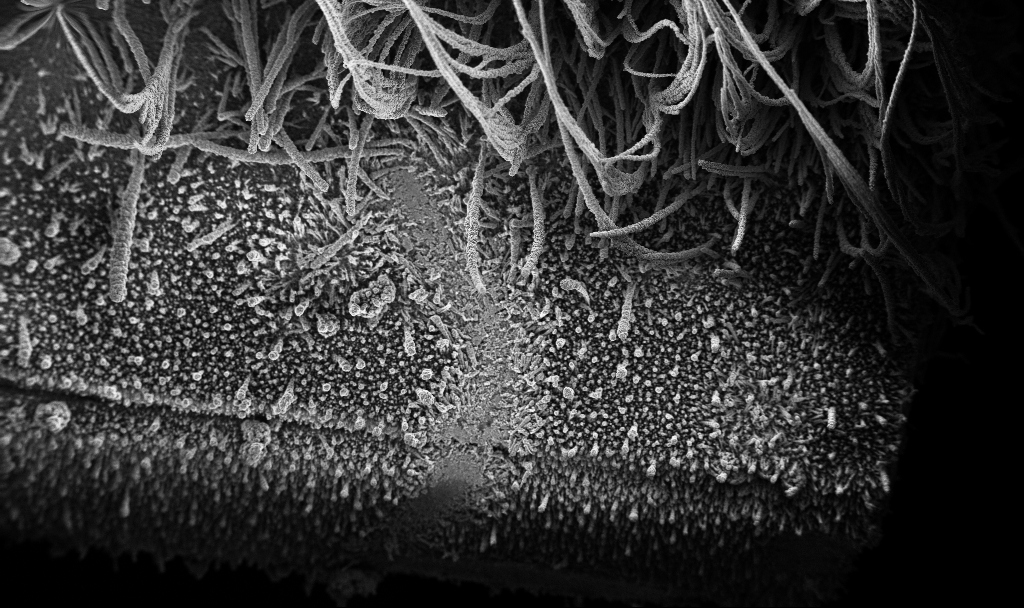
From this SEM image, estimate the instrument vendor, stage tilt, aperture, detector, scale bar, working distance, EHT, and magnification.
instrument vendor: Zeiss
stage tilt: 0°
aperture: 30 µm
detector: InLens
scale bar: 100000 nm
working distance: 3.7 mm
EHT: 3 kV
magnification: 0.15 K X